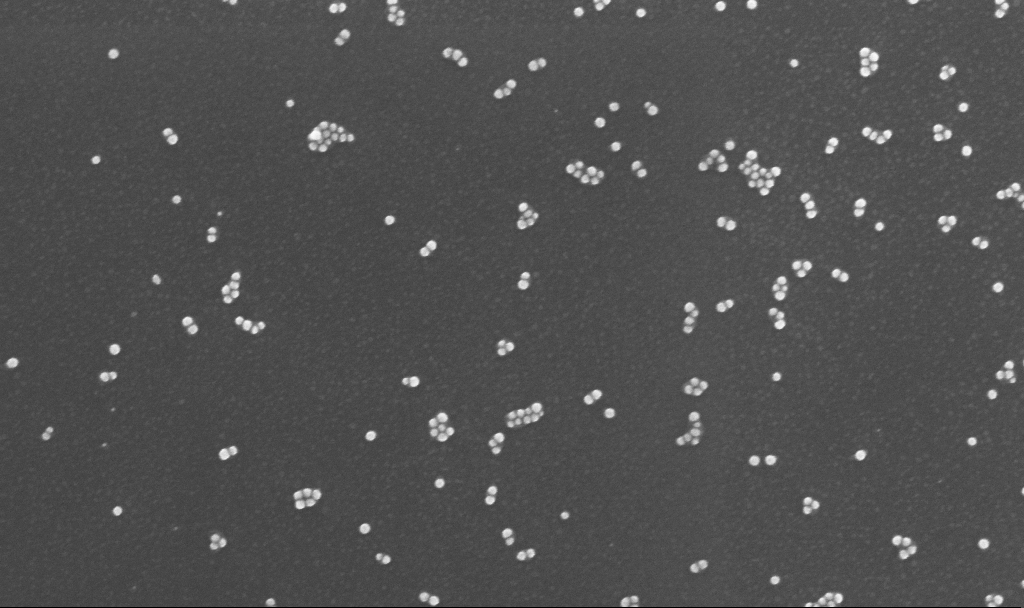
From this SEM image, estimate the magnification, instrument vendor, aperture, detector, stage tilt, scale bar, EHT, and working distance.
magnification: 150 K X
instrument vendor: Zeiss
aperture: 30 µm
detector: InLens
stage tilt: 0°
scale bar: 200 nm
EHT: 10 kV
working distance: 3.2 mm